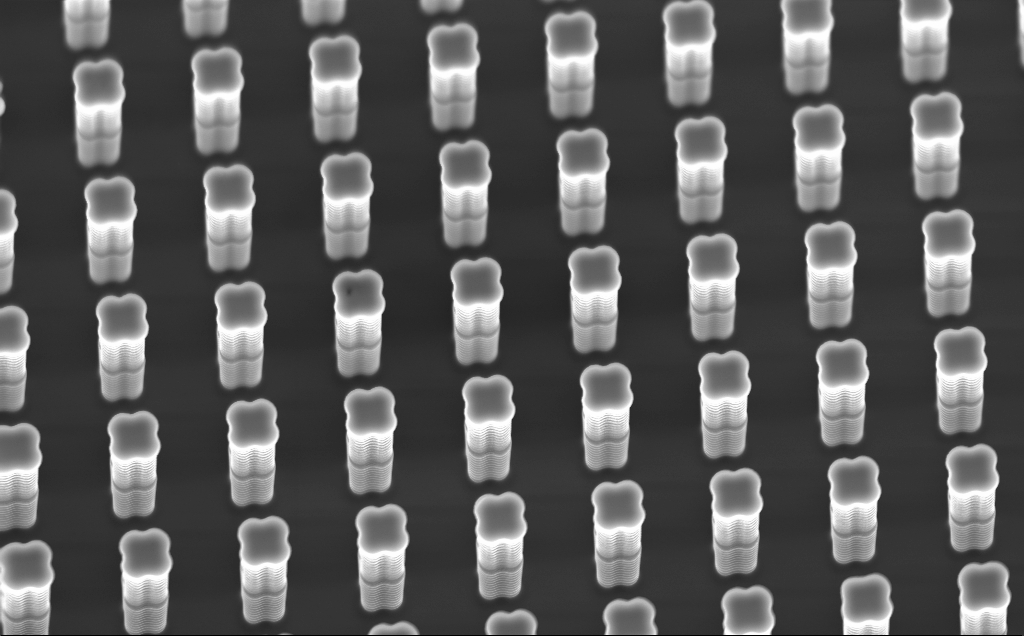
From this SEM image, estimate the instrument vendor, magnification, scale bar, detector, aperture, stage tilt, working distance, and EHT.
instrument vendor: Zeiss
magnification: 4.36 K X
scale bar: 10000 nm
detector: InLens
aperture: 120 µm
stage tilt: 30.3°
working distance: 9 mm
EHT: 10 kV